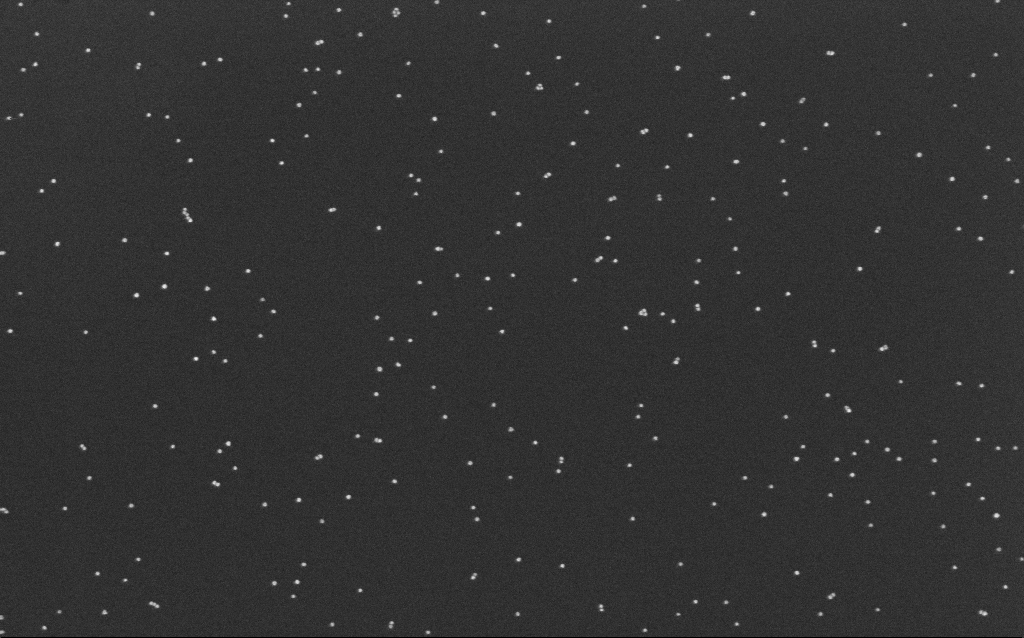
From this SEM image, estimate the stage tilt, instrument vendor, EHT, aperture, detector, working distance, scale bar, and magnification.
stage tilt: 0°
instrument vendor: Zeiss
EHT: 10 kV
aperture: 30 µm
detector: InLens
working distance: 6.6 mm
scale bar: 200 nm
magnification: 100 K X